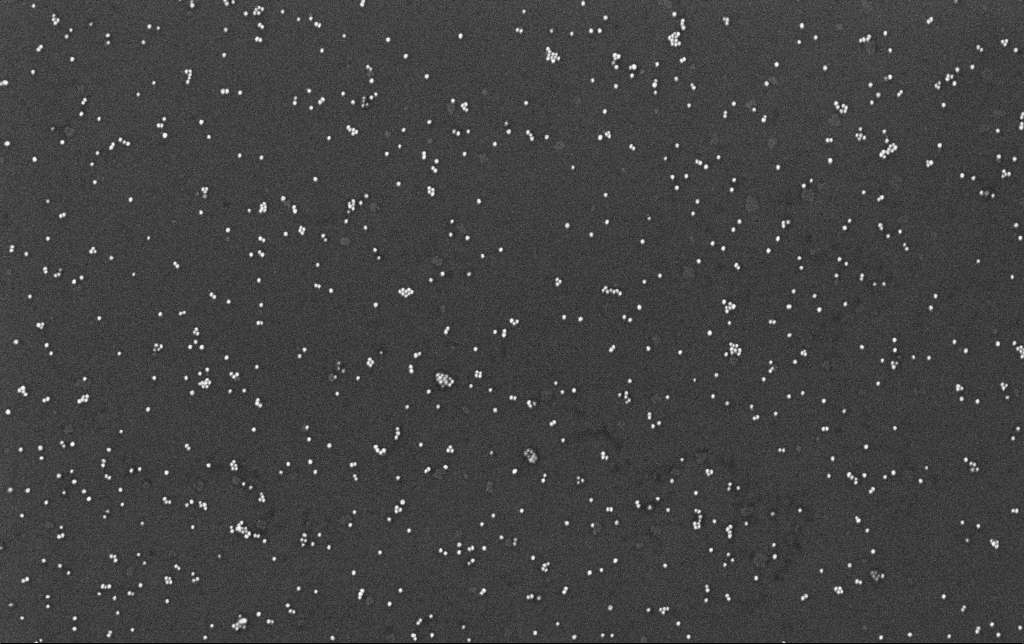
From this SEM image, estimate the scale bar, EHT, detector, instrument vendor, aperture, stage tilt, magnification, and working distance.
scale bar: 200 nm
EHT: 10 kV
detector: InLens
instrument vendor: Zeiss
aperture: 30 µm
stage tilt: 0°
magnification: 100 K X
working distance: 3.4 mm